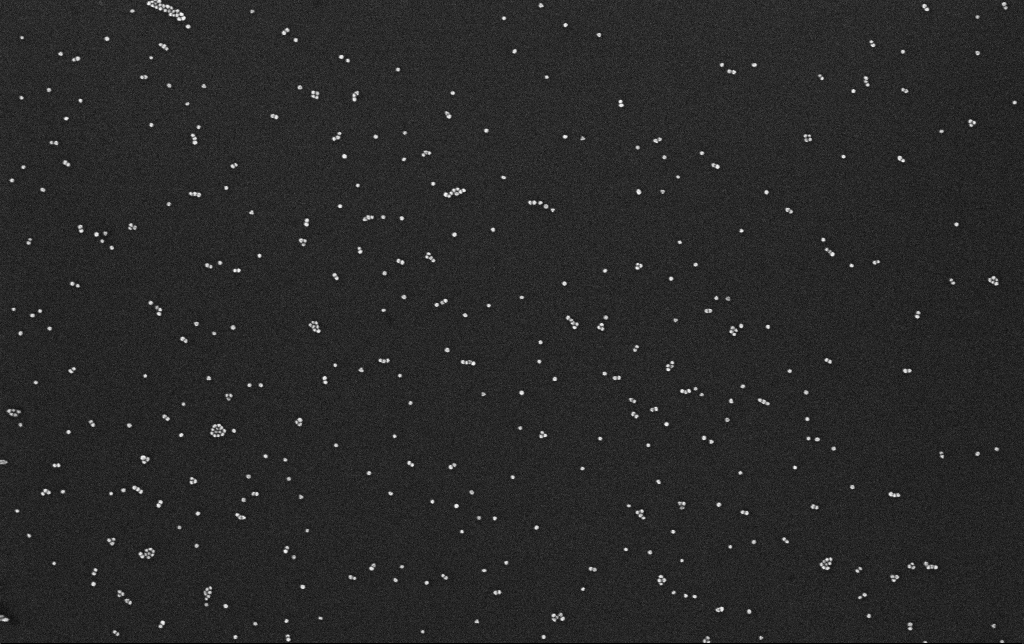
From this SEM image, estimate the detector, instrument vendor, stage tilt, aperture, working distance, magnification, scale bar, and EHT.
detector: InLens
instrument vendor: Zeiss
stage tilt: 0°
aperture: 30 µm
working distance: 3.1 mm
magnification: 100 K X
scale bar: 200 nm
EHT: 10 kV